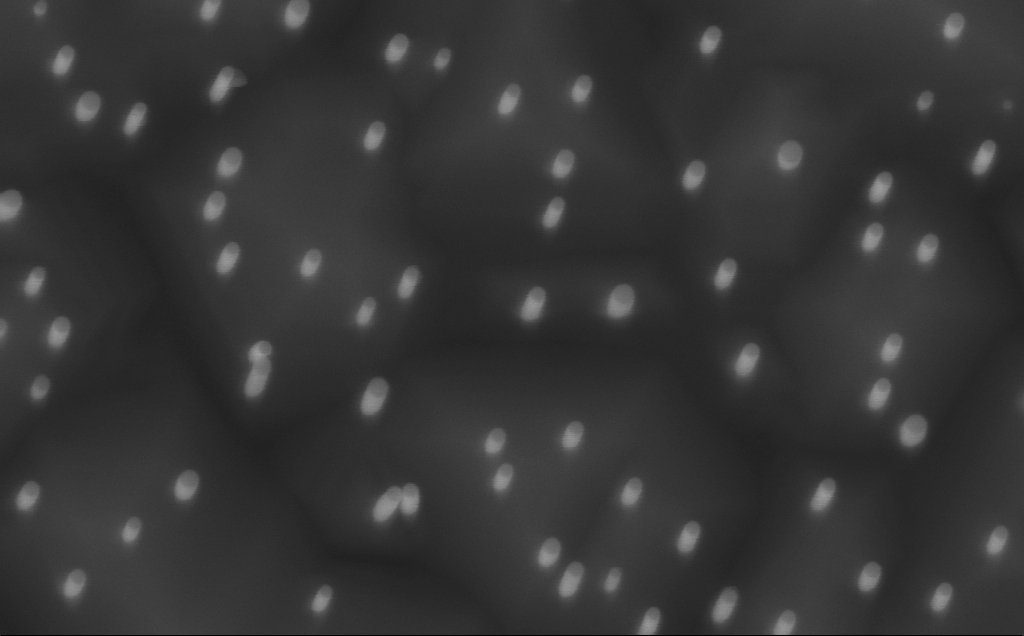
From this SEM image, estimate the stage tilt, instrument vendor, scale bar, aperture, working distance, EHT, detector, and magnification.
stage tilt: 0°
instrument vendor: Zeiss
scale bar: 100 nm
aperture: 30 µm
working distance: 5 mm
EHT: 10 kV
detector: InLens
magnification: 150 K X